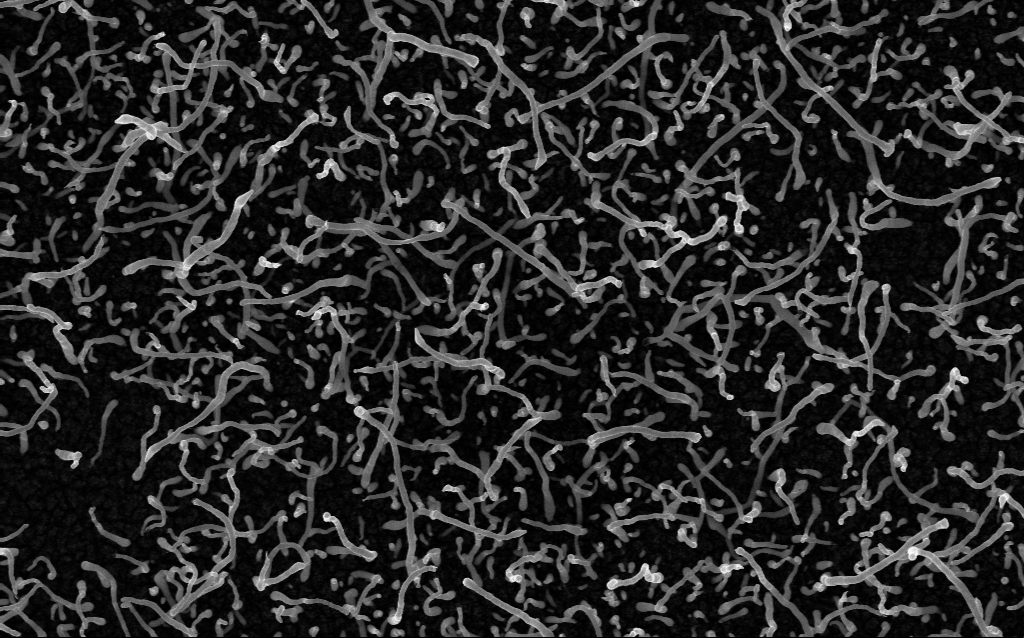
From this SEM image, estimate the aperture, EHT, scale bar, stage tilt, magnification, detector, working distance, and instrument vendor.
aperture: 30 µm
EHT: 5 kV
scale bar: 1000 nm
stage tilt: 0°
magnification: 50 K X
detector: InLens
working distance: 2 mm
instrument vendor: Zeiss